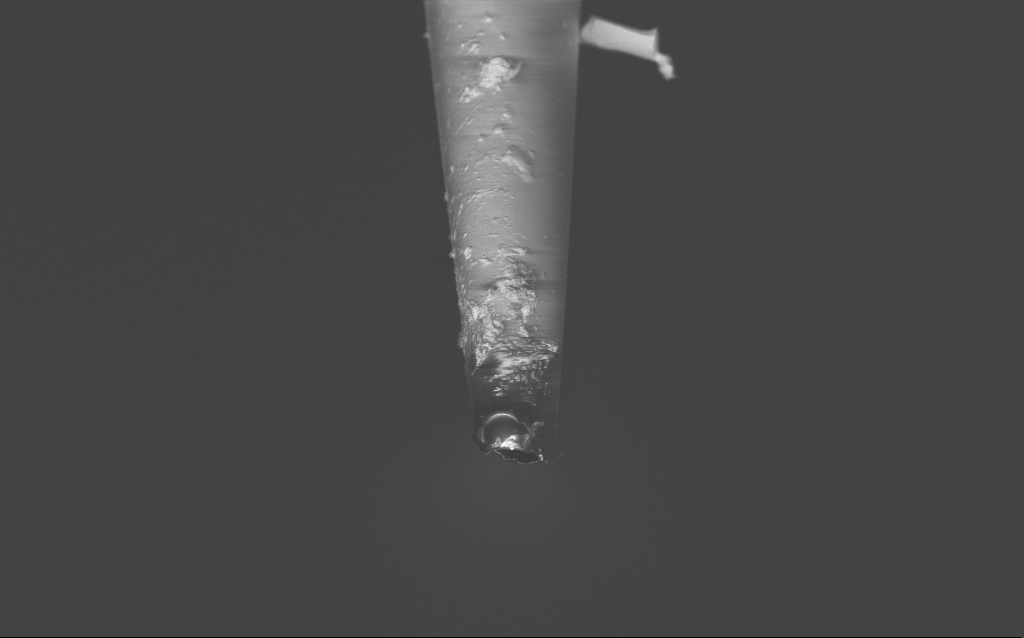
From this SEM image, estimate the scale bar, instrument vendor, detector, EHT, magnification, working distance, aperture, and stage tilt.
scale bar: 10000 nm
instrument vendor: Zeiss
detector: InLens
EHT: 2 kV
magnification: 5 K X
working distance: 6 mm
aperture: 30 µm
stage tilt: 45°